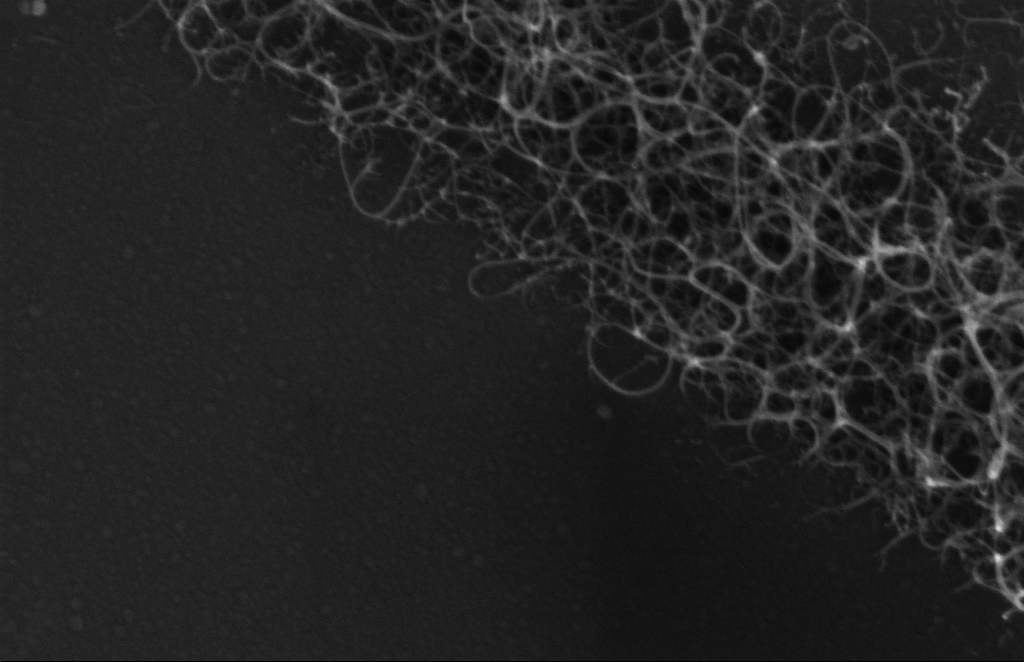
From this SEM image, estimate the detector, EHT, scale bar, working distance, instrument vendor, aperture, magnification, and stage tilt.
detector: InLens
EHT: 5 kV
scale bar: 200 nm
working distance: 5 mm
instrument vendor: Zeiss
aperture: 20 µm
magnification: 228.2 K X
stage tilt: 0°